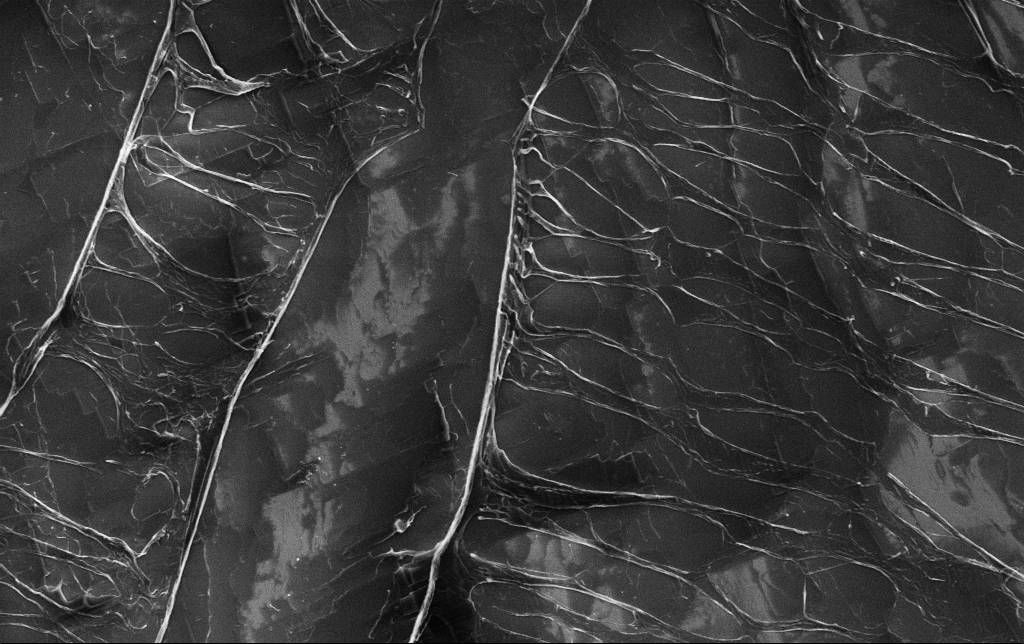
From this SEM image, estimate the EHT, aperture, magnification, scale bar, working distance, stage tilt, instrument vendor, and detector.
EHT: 5 kV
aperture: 30 µm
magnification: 3.53 K X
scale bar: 20000 nm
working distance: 3.1 mm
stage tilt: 0°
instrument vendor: Zeiss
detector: InLens